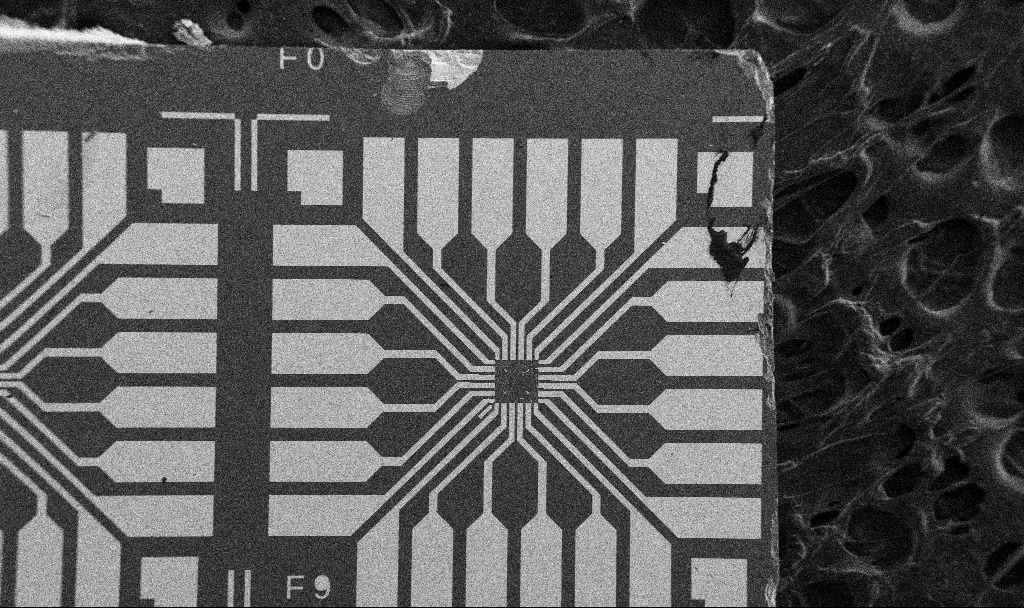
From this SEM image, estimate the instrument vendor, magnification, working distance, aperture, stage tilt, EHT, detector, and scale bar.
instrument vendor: Zeiss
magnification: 0.1 K X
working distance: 10.7 mm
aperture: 30 µm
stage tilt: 0°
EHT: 5 kV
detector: SE2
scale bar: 200000 nm